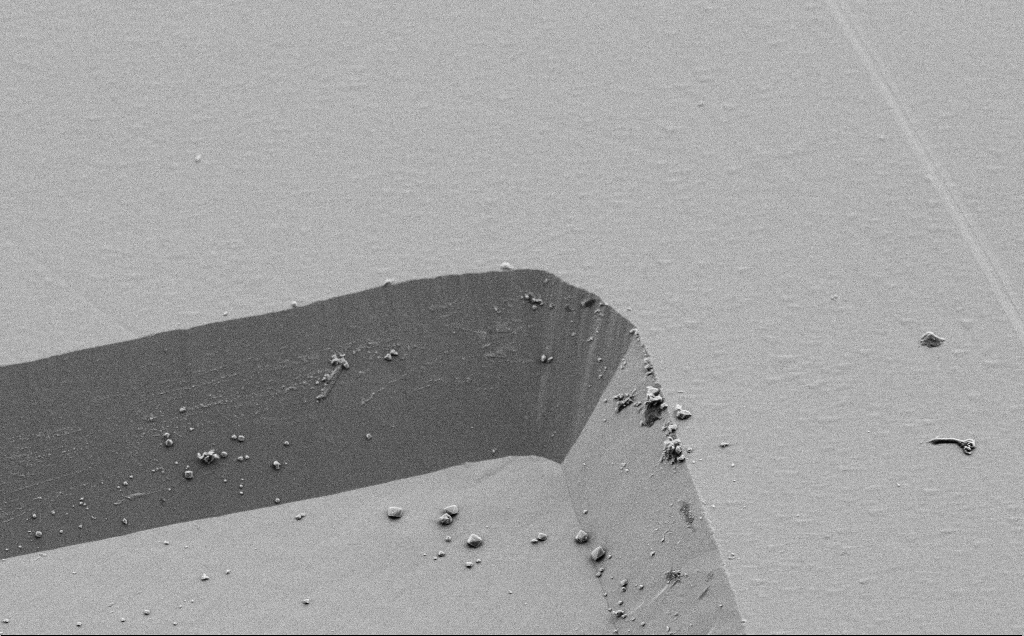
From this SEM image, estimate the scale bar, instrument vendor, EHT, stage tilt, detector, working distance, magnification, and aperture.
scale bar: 10000 nm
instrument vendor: Zeiss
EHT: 3 kV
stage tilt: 45°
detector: SE2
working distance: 9 mm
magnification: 3.48 K X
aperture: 30 µm